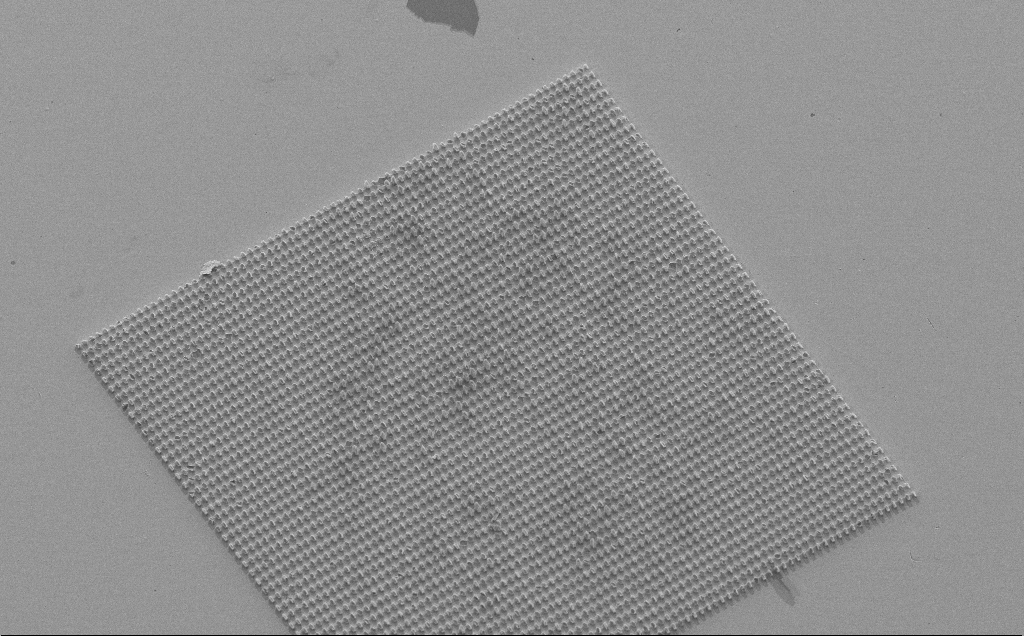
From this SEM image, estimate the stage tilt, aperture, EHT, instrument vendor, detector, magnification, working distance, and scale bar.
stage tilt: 32.4°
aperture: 30 µm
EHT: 10 kV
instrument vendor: Zeiss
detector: SE2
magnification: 0.76 K X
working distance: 10 mm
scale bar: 20000 nm